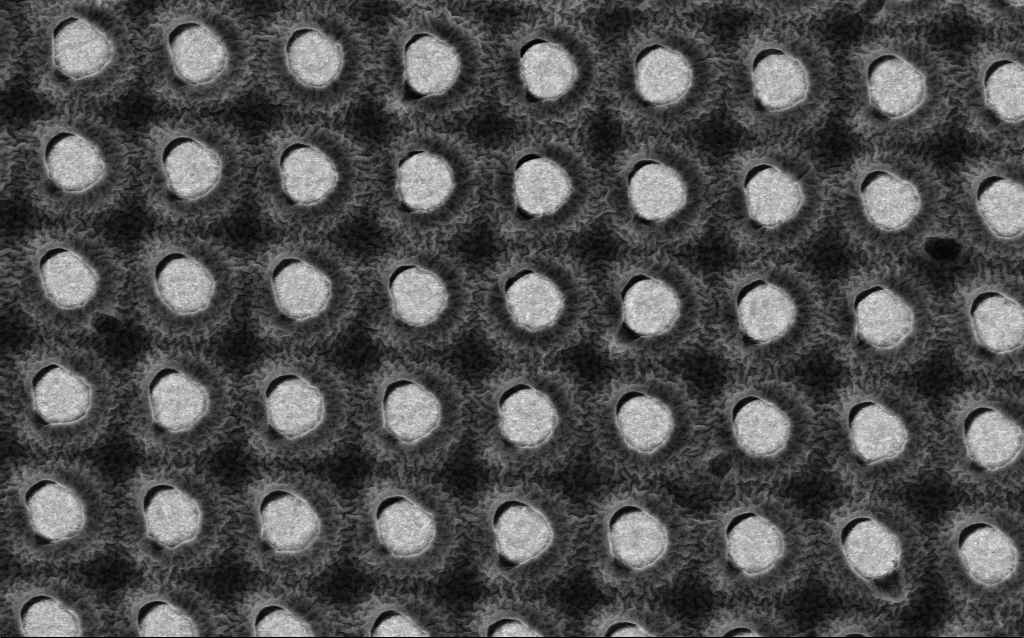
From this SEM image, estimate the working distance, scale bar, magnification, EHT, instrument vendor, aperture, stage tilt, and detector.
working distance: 3.1 mm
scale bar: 200 nm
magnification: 86.43 K X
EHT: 2 kV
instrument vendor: Zeiss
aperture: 30 µm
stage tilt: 0°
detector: InLens